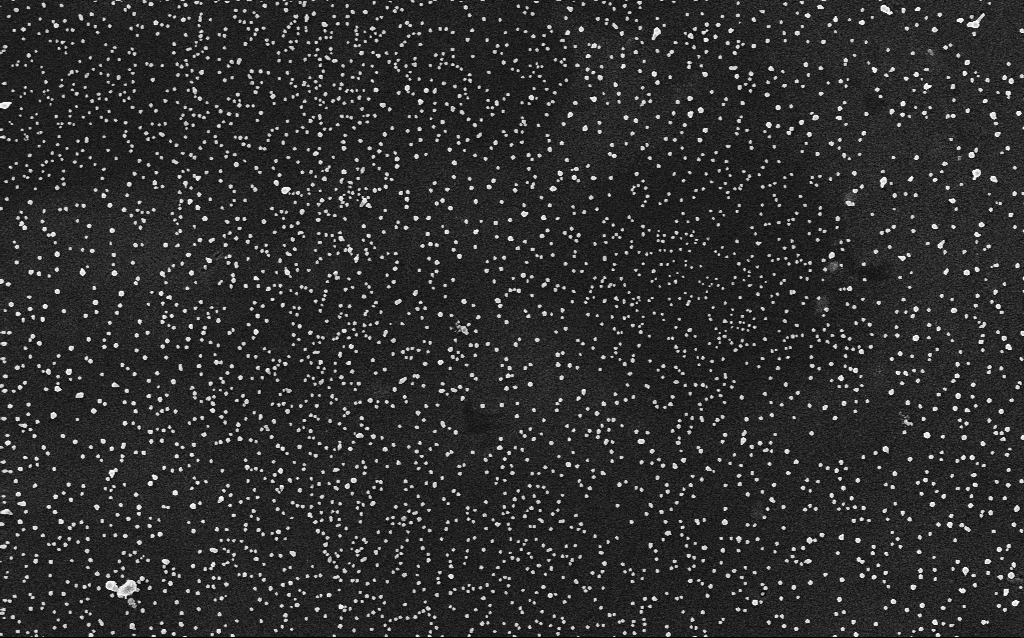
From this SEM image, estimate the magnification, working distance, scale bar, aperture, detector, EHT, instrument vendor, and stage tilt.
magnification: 10 K X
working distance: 2.8 mm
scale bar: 2000 nm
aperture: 30 µm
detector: InLens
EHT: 5 kV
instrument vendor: Zeiss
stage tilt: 0°